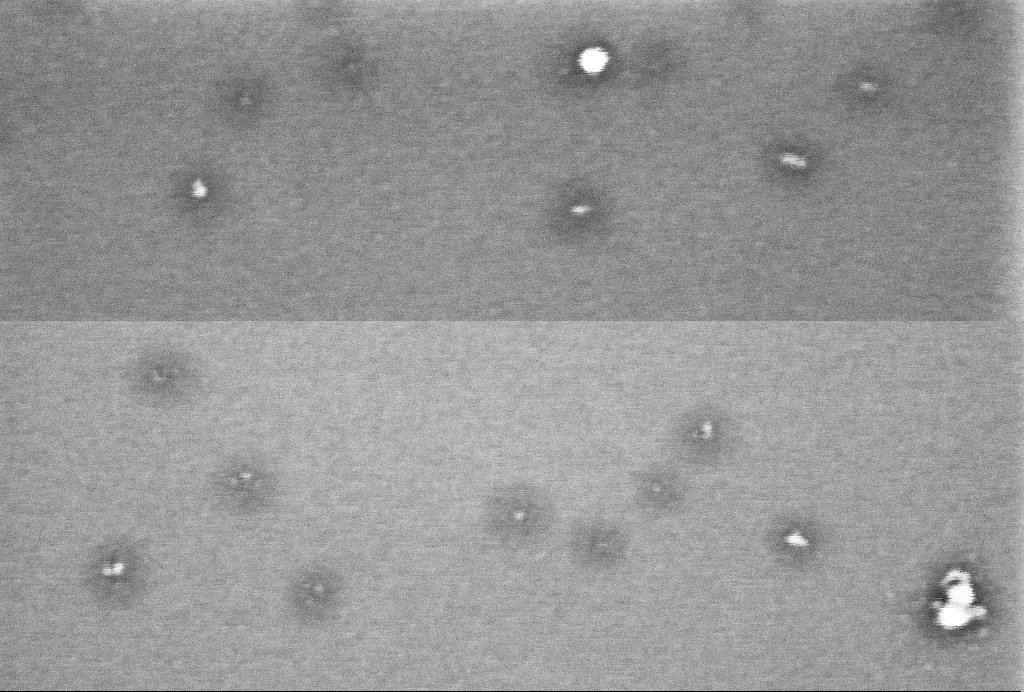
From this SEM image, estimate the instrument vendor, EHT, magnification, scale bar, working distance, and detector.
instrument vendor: Zeiss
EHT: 1 kV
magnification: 305.73 K X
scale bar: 100 nm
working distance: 3.4 mm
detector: InLens